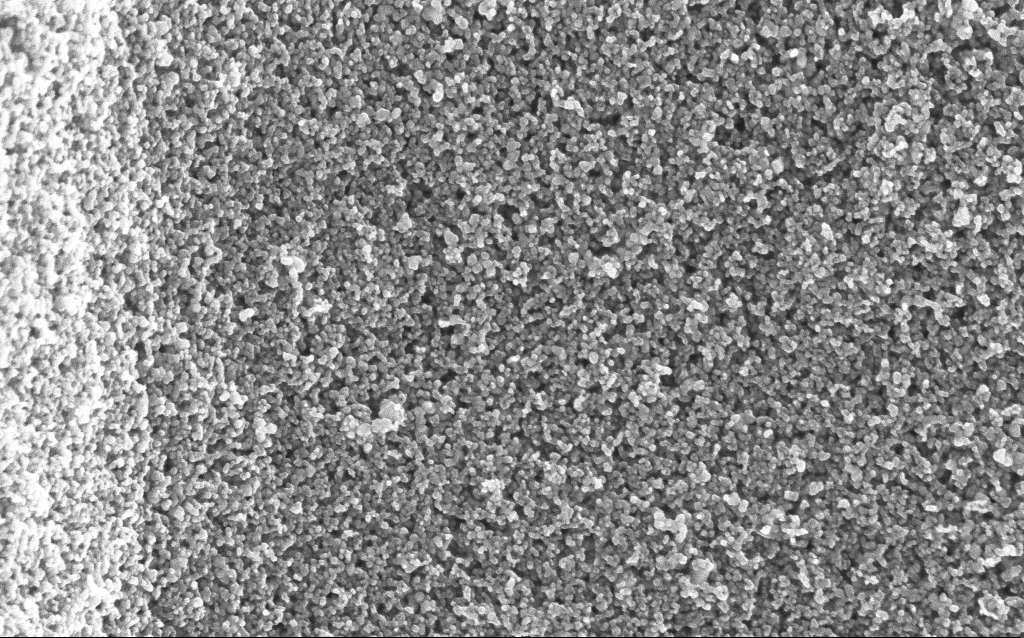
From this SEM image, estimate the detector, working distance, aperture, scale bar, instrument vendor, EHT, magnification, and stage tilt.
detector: InLens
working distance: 6.4 mm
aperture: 30 µm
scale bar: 200 nm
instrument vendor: Zeiss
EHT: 5 kV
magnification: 75.49 K X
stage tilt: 0°